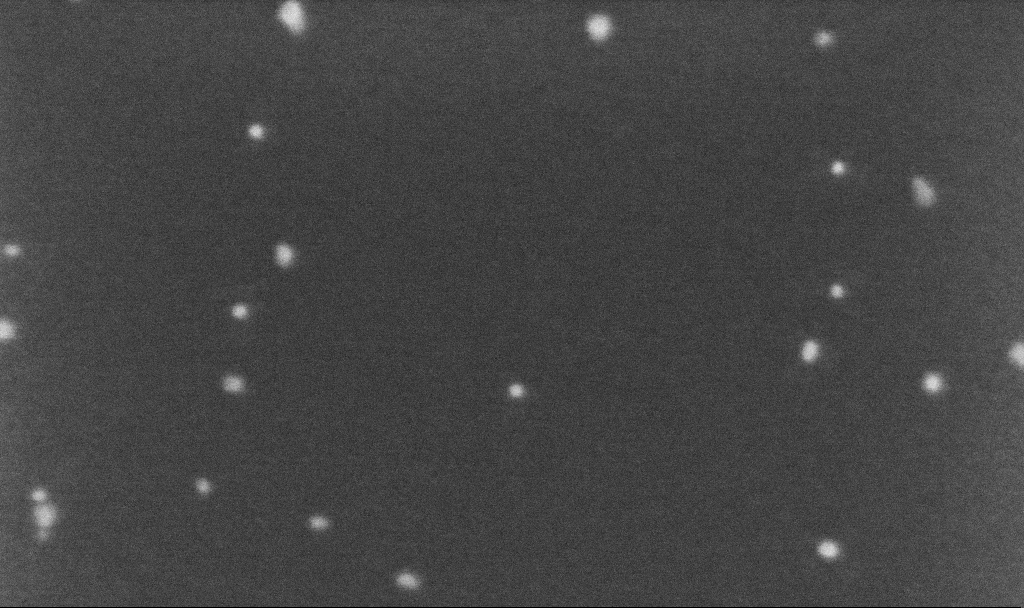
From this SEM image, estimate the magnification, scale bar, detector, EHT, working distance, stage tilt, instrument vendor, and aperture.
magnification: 500 K X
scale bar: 100 nm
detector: InLens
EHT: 5 kV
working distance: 3.2 mm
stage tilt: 0°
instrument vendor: Zeiss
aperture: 30 µm